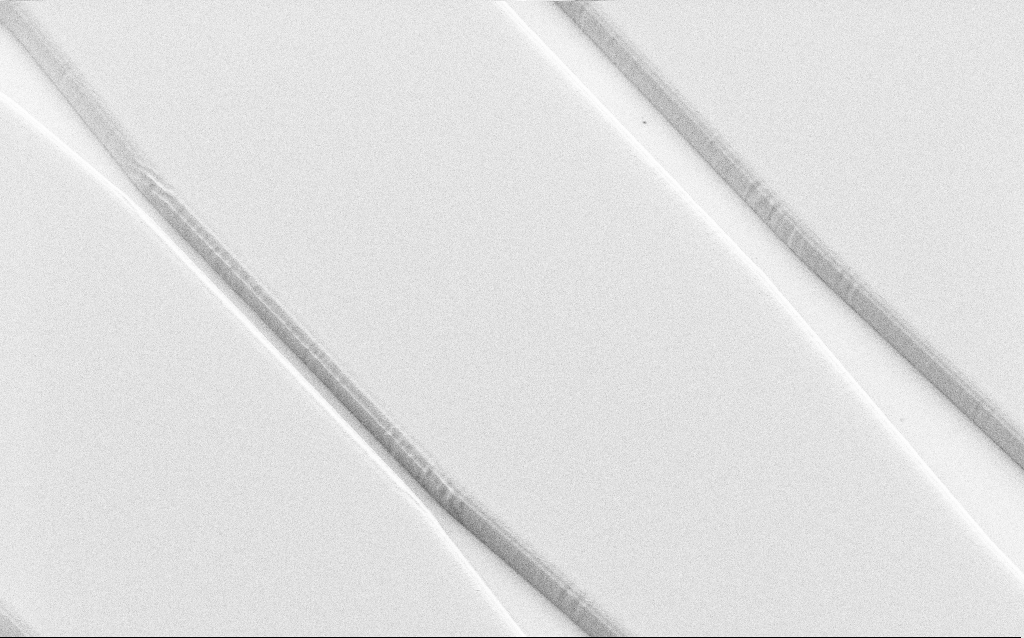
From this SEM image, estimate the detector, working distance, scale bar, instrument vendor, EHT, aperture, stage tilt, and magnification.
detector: SE2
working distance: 6 mm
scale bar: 20000 nm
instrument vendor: Zeiss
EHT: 1 kV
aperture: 30 µm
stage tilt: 36°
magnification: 1.57 K X